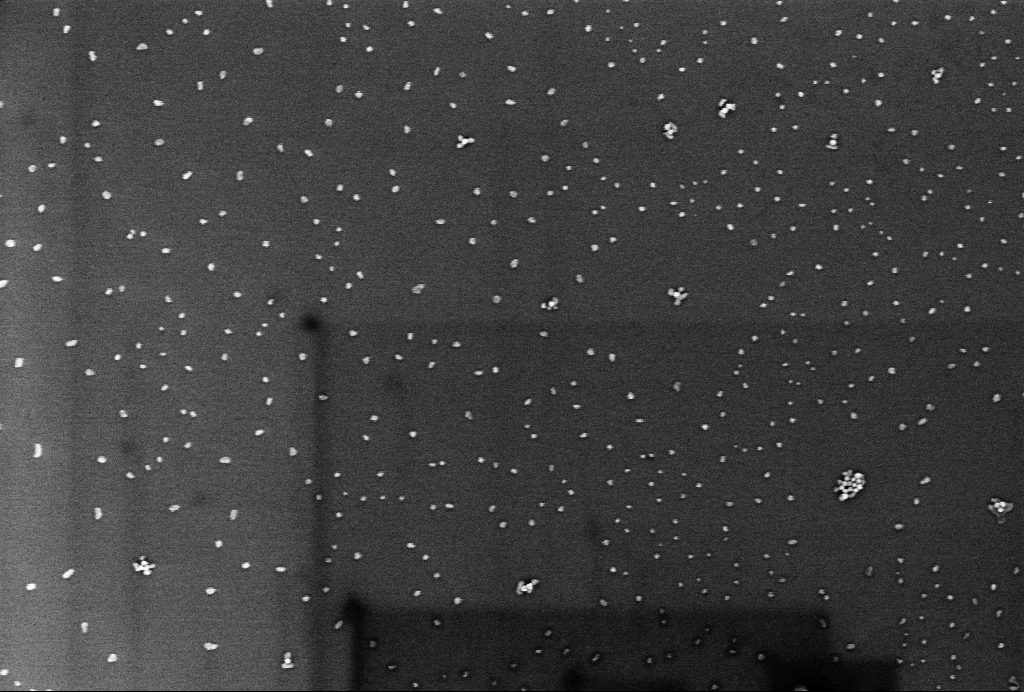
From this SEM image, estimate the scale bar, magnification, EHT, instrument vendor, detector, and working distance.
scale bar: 200 nm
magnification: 86.59 K X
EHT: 1 kV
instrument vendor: Zeiss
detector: InLens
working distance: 3.1 mm